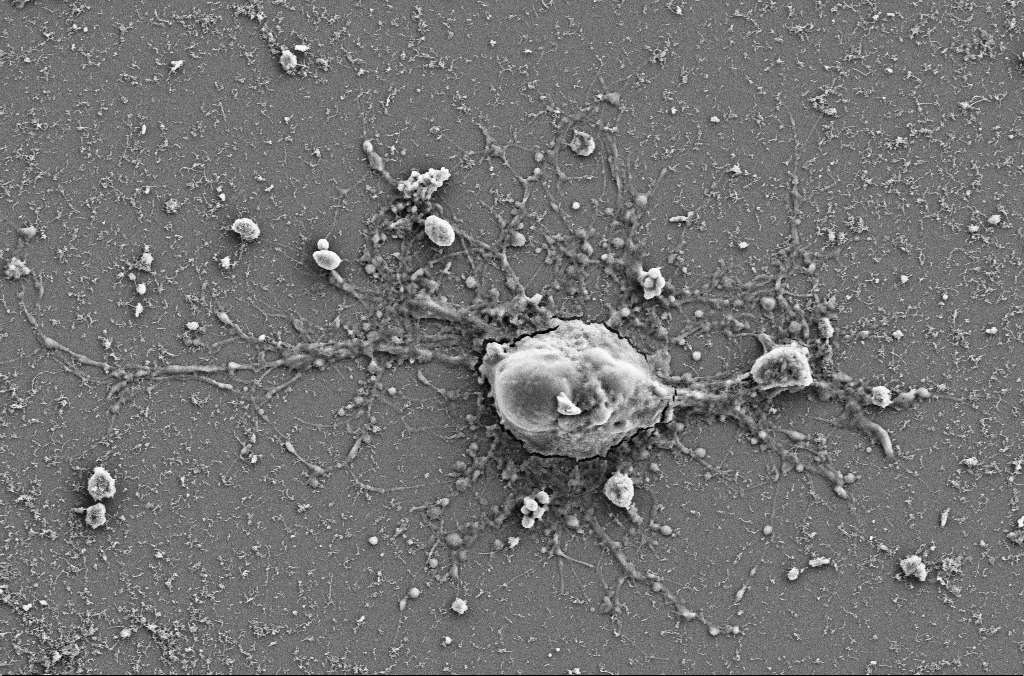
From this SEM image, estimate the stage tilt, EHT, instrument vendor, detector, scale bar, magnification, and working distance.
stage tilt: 0°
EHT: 5 kV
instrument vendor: Zeiss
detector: SE2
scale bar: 10000 nm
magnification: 5 K X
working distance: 4 mm